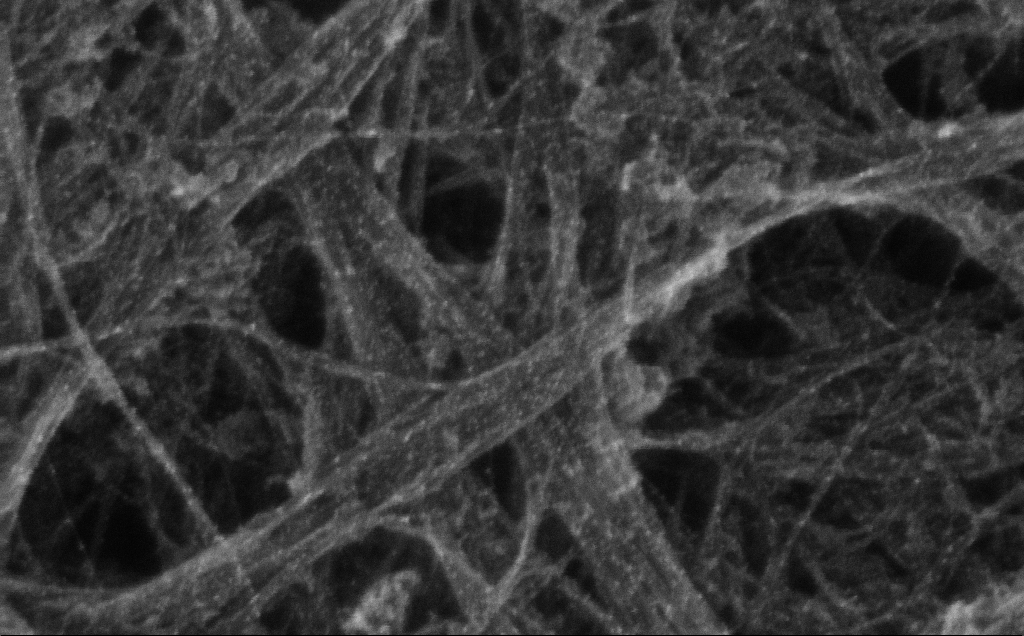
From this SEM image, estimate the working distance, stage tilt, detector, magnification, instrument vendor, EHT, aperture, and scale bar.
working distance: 3 mm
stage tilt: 0°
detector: InLens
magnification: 250.02 K X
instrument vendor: Zeiss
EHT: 10 kV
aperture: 30 µm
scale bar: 200 nm